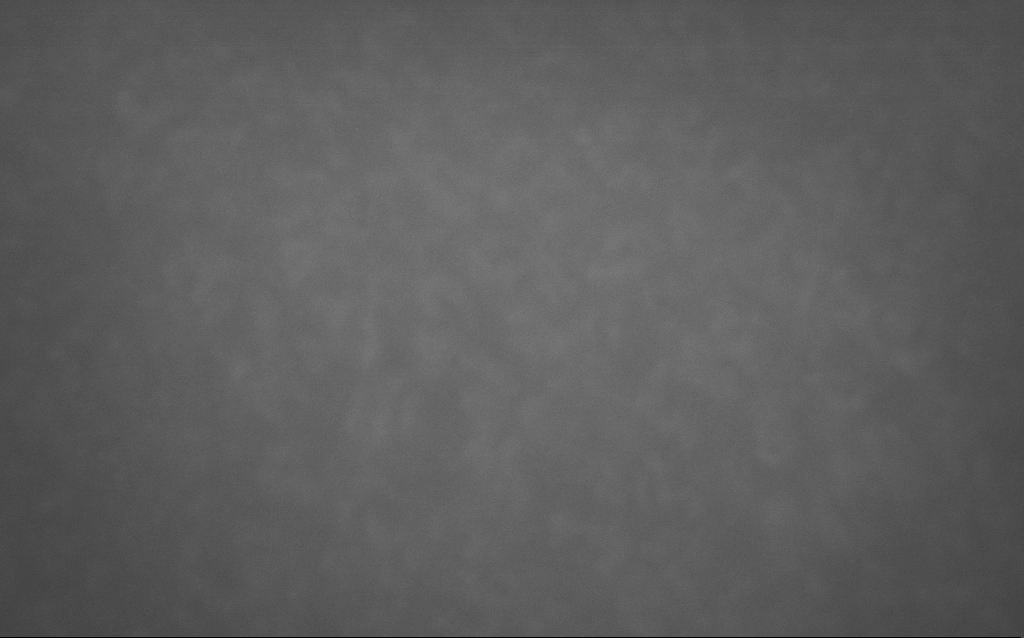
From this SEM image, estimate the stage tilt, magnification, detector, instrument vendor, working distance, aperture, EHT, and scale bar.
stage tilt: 0°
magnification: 1185.18 K X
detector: InLens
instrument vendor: Zeiss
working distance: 3 mm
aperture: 30 µm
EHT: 10 kV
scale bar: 20 nm